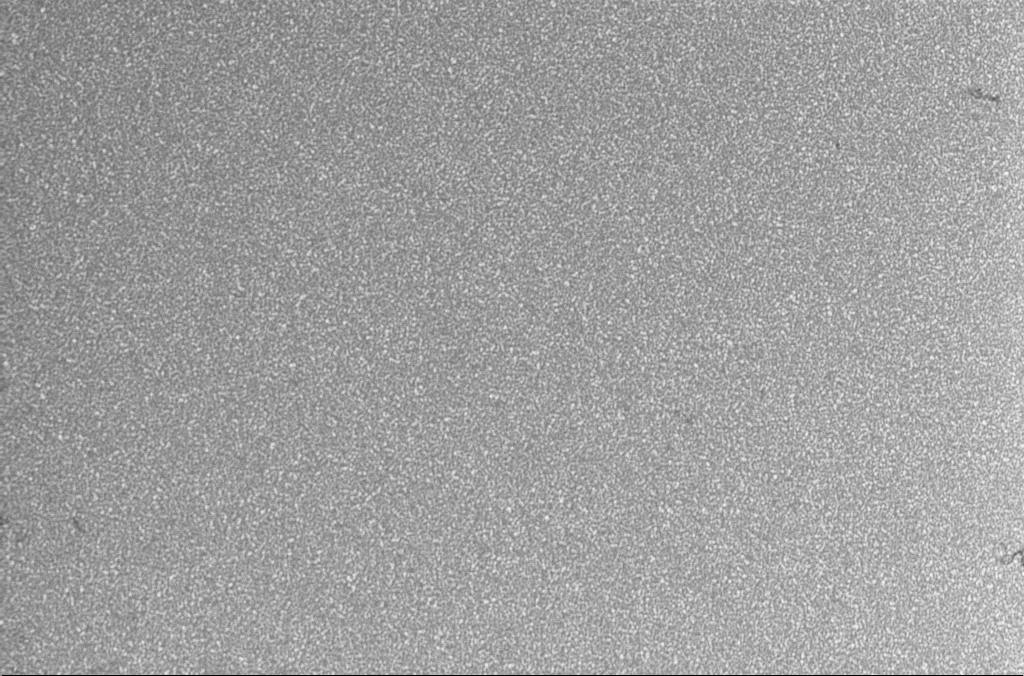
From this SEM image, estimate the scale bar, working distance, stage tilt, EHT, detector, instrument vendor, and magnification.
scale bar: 10000 nm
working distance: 1.9 mm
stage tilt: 0°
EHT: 5 kV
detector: InLens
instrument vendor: Zeiss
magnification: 5 K X